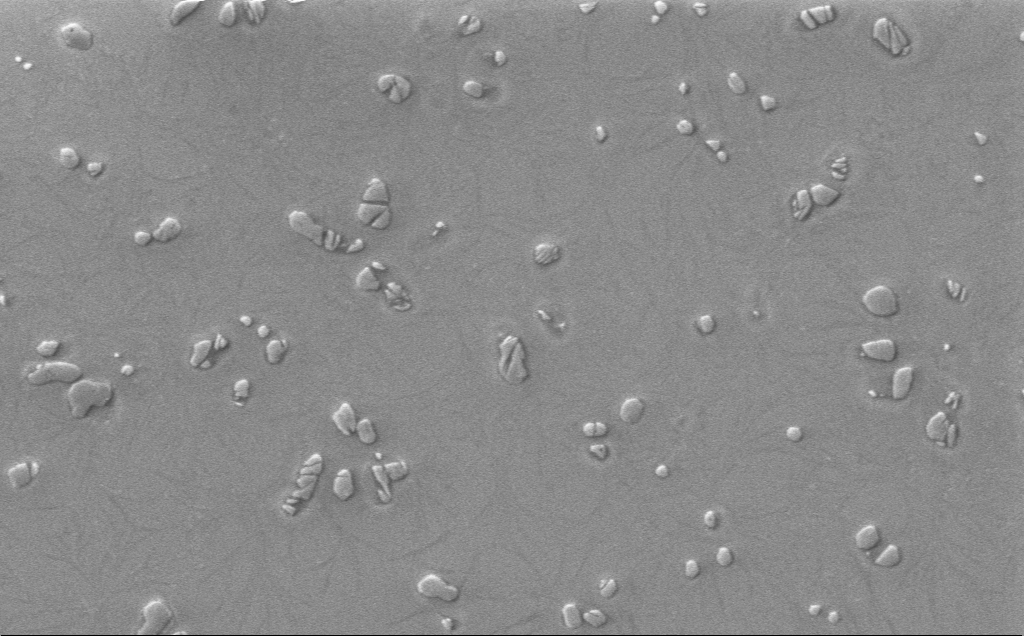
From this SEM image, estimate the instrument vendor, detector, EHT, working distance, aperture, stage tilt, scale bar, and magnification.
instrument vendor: Zeiss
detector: InLens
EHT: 1 kV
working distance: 4 mm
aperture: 30 µm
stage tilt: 0°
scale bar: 10000 nm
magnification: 5.54 K X